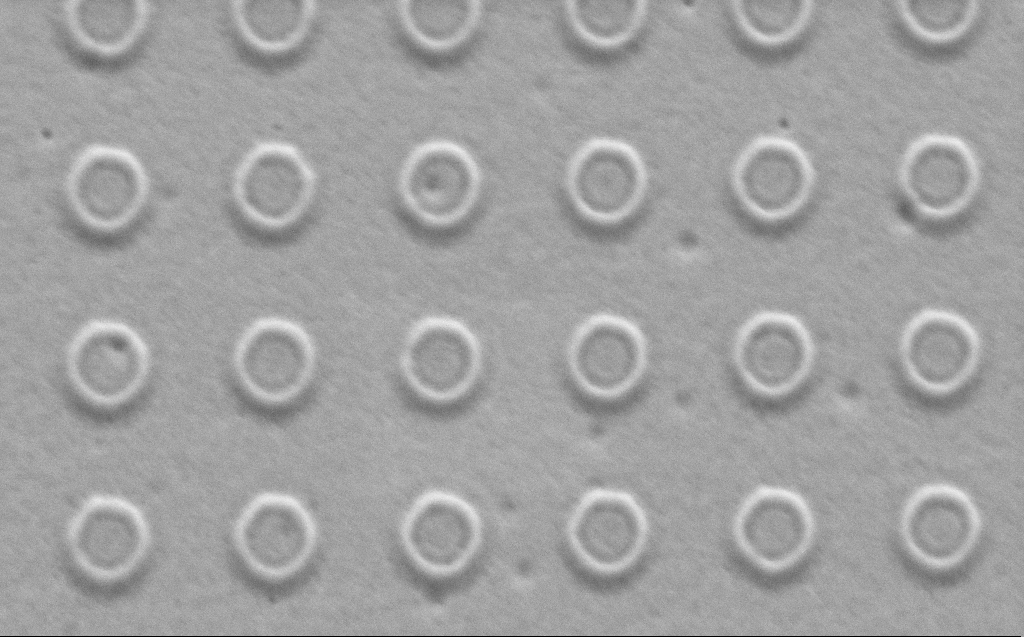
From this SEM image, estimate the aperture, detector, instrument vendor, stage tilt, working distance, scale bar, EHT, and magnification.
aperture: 30 µm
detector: SE2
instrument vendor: Zeiss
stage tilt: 41.9°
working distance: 9 mm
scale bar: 1000 nm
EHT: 3 kV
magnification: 50.82 K X